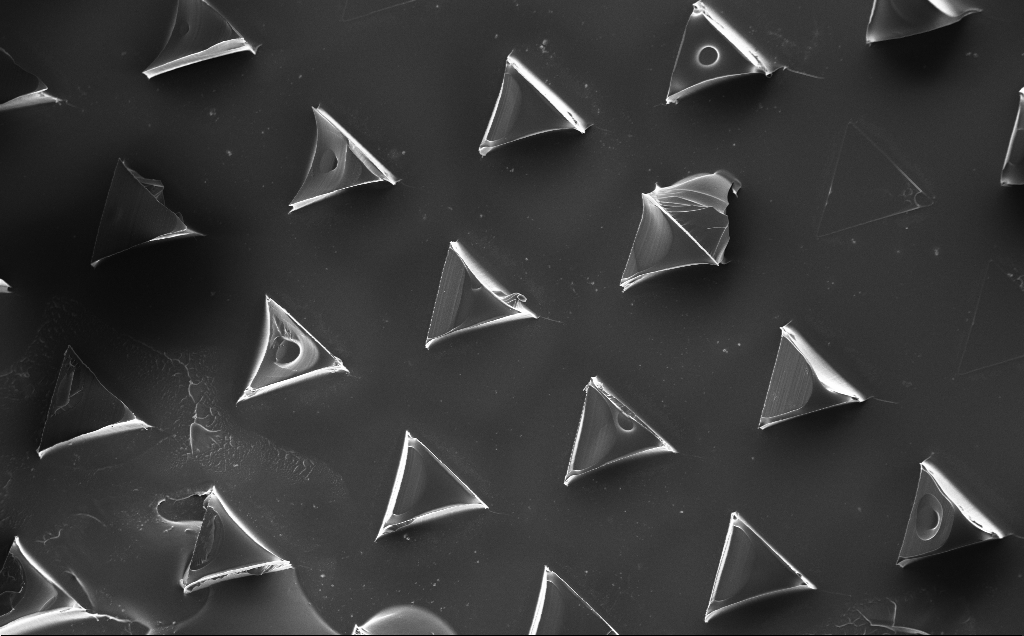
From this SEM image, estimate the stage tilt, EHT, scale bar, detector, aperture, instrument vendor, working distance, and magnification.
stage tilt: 0°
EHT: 10 kV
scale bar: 100000 nm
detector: InLens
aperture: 30 µm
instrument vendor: Zeiss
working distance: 9 mm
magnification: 0.158 K X